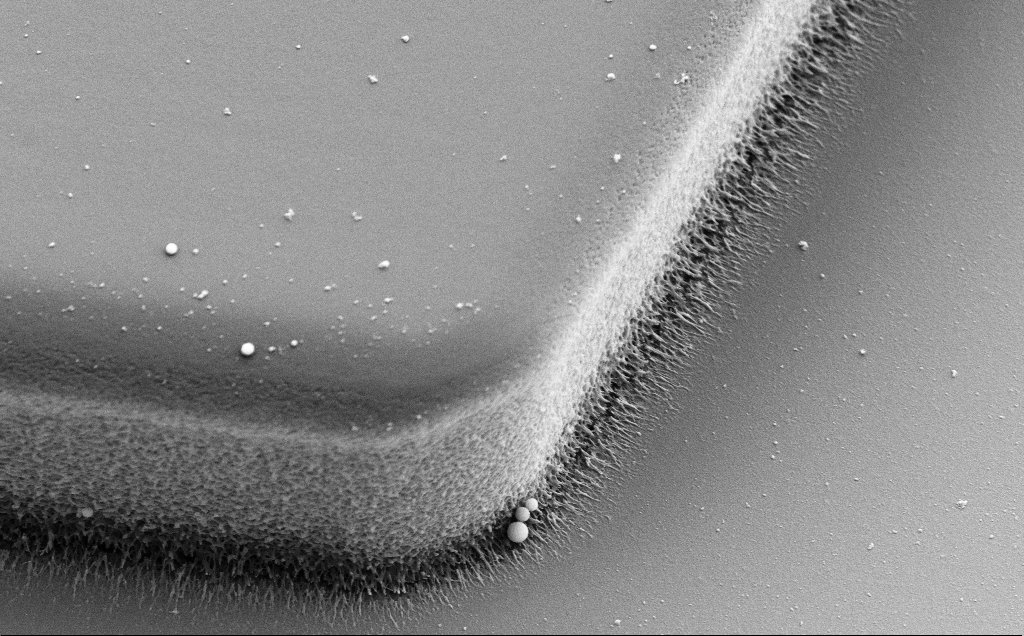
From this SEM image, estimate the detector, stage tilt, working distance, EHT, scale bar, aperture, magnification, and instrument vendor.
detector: SE2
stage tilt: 30°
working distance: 8 mm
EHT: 5 kV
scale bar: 1000 nm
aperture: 30 µm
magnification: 14.06 K X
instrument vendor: Zeiss